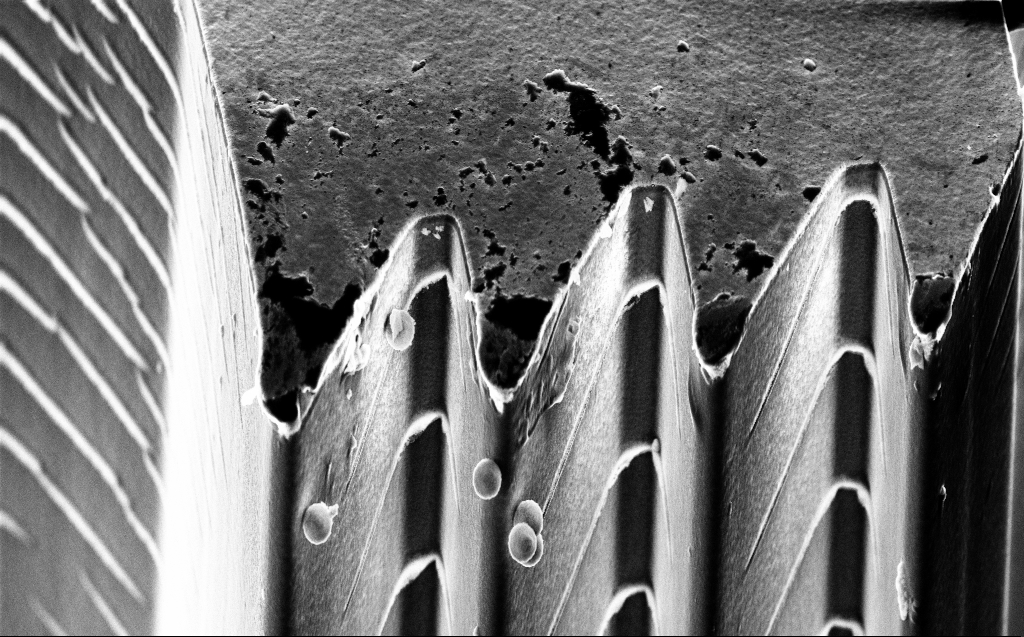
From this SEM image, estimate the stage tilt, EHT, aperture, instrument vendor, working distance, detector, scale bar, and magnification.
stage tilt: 45°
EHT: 10 kV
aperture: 30 µm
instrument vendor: Zeiss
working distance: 5 mm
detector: InLens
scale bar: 10000 nm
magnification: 7.12 K X